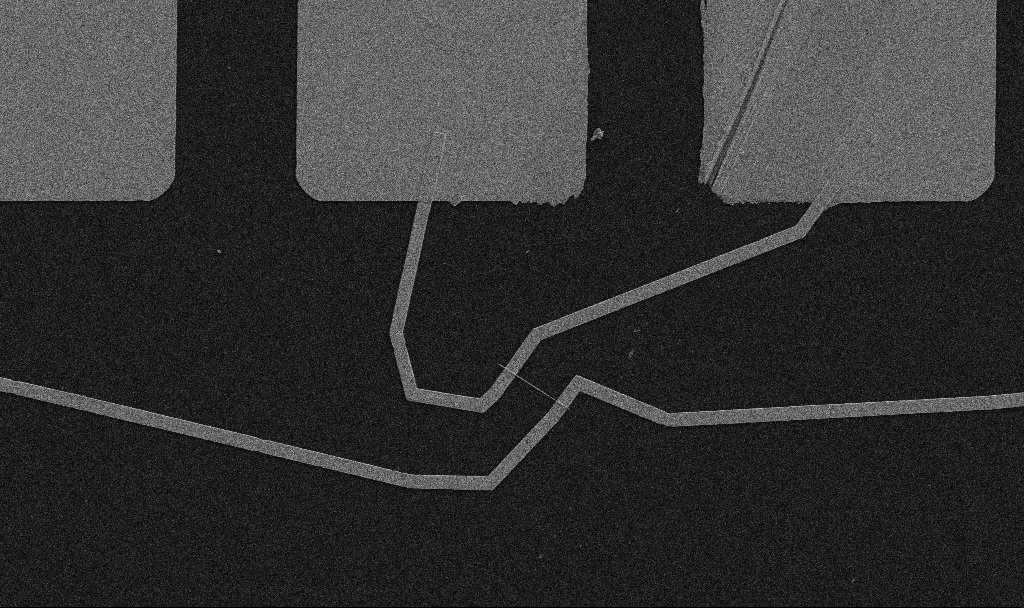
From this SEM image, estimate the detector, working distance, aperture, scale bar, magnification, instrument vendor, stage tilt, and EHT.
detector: SE2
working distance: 10.7 mm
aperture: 30 µm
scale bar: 10000 nm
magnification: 5 K X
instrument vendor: Zeiss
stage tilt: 0°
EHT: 5 kV